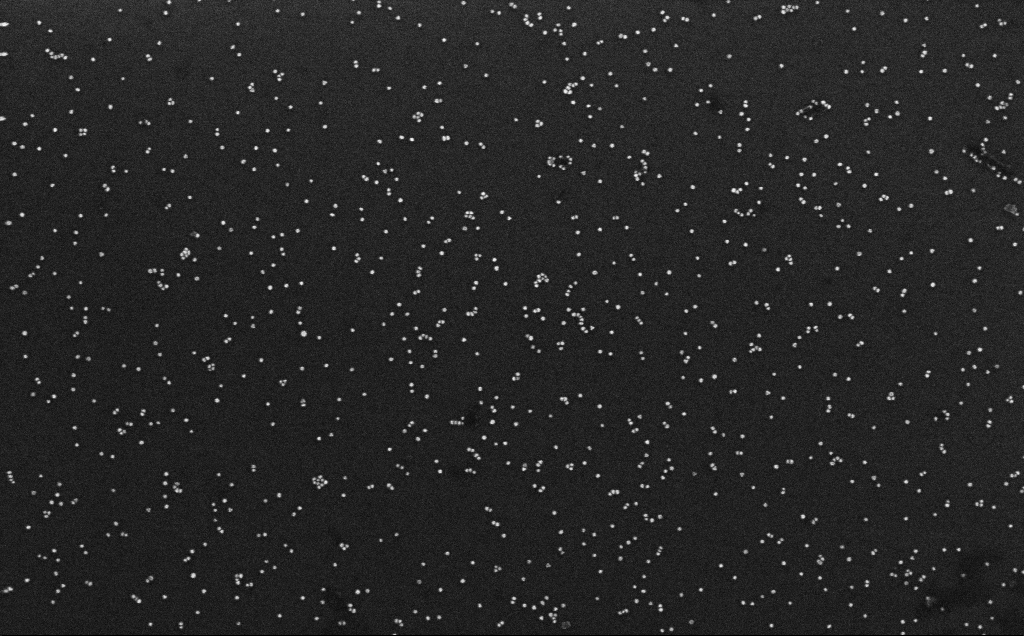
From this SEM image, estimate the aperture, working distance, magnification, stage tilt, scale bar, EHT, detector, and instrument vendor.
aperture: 30 µm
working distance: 3.3 mm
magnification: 100 K X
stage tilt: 0°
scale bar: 200 nm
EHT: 10 kV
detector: InLens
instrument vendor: Zeiss